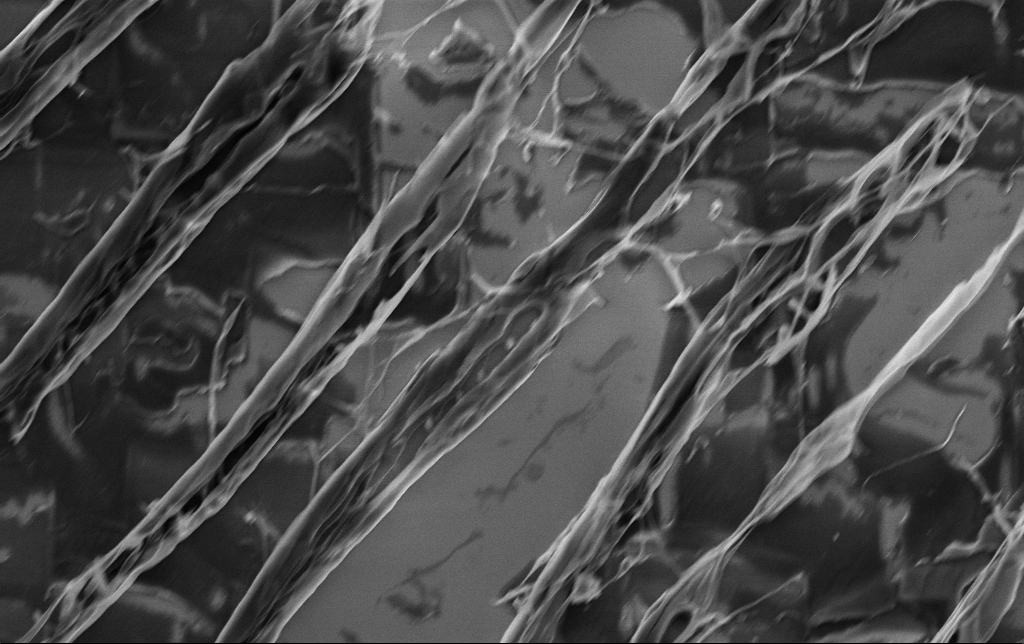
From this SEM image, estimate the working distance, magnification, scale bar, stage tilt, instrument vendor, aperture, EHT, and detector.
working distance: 3.1 mm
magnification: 11.14 K X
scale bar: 2000 nm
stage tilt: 0°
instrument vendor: Zeiss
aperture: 30 µm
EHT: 3 kV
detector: InLens